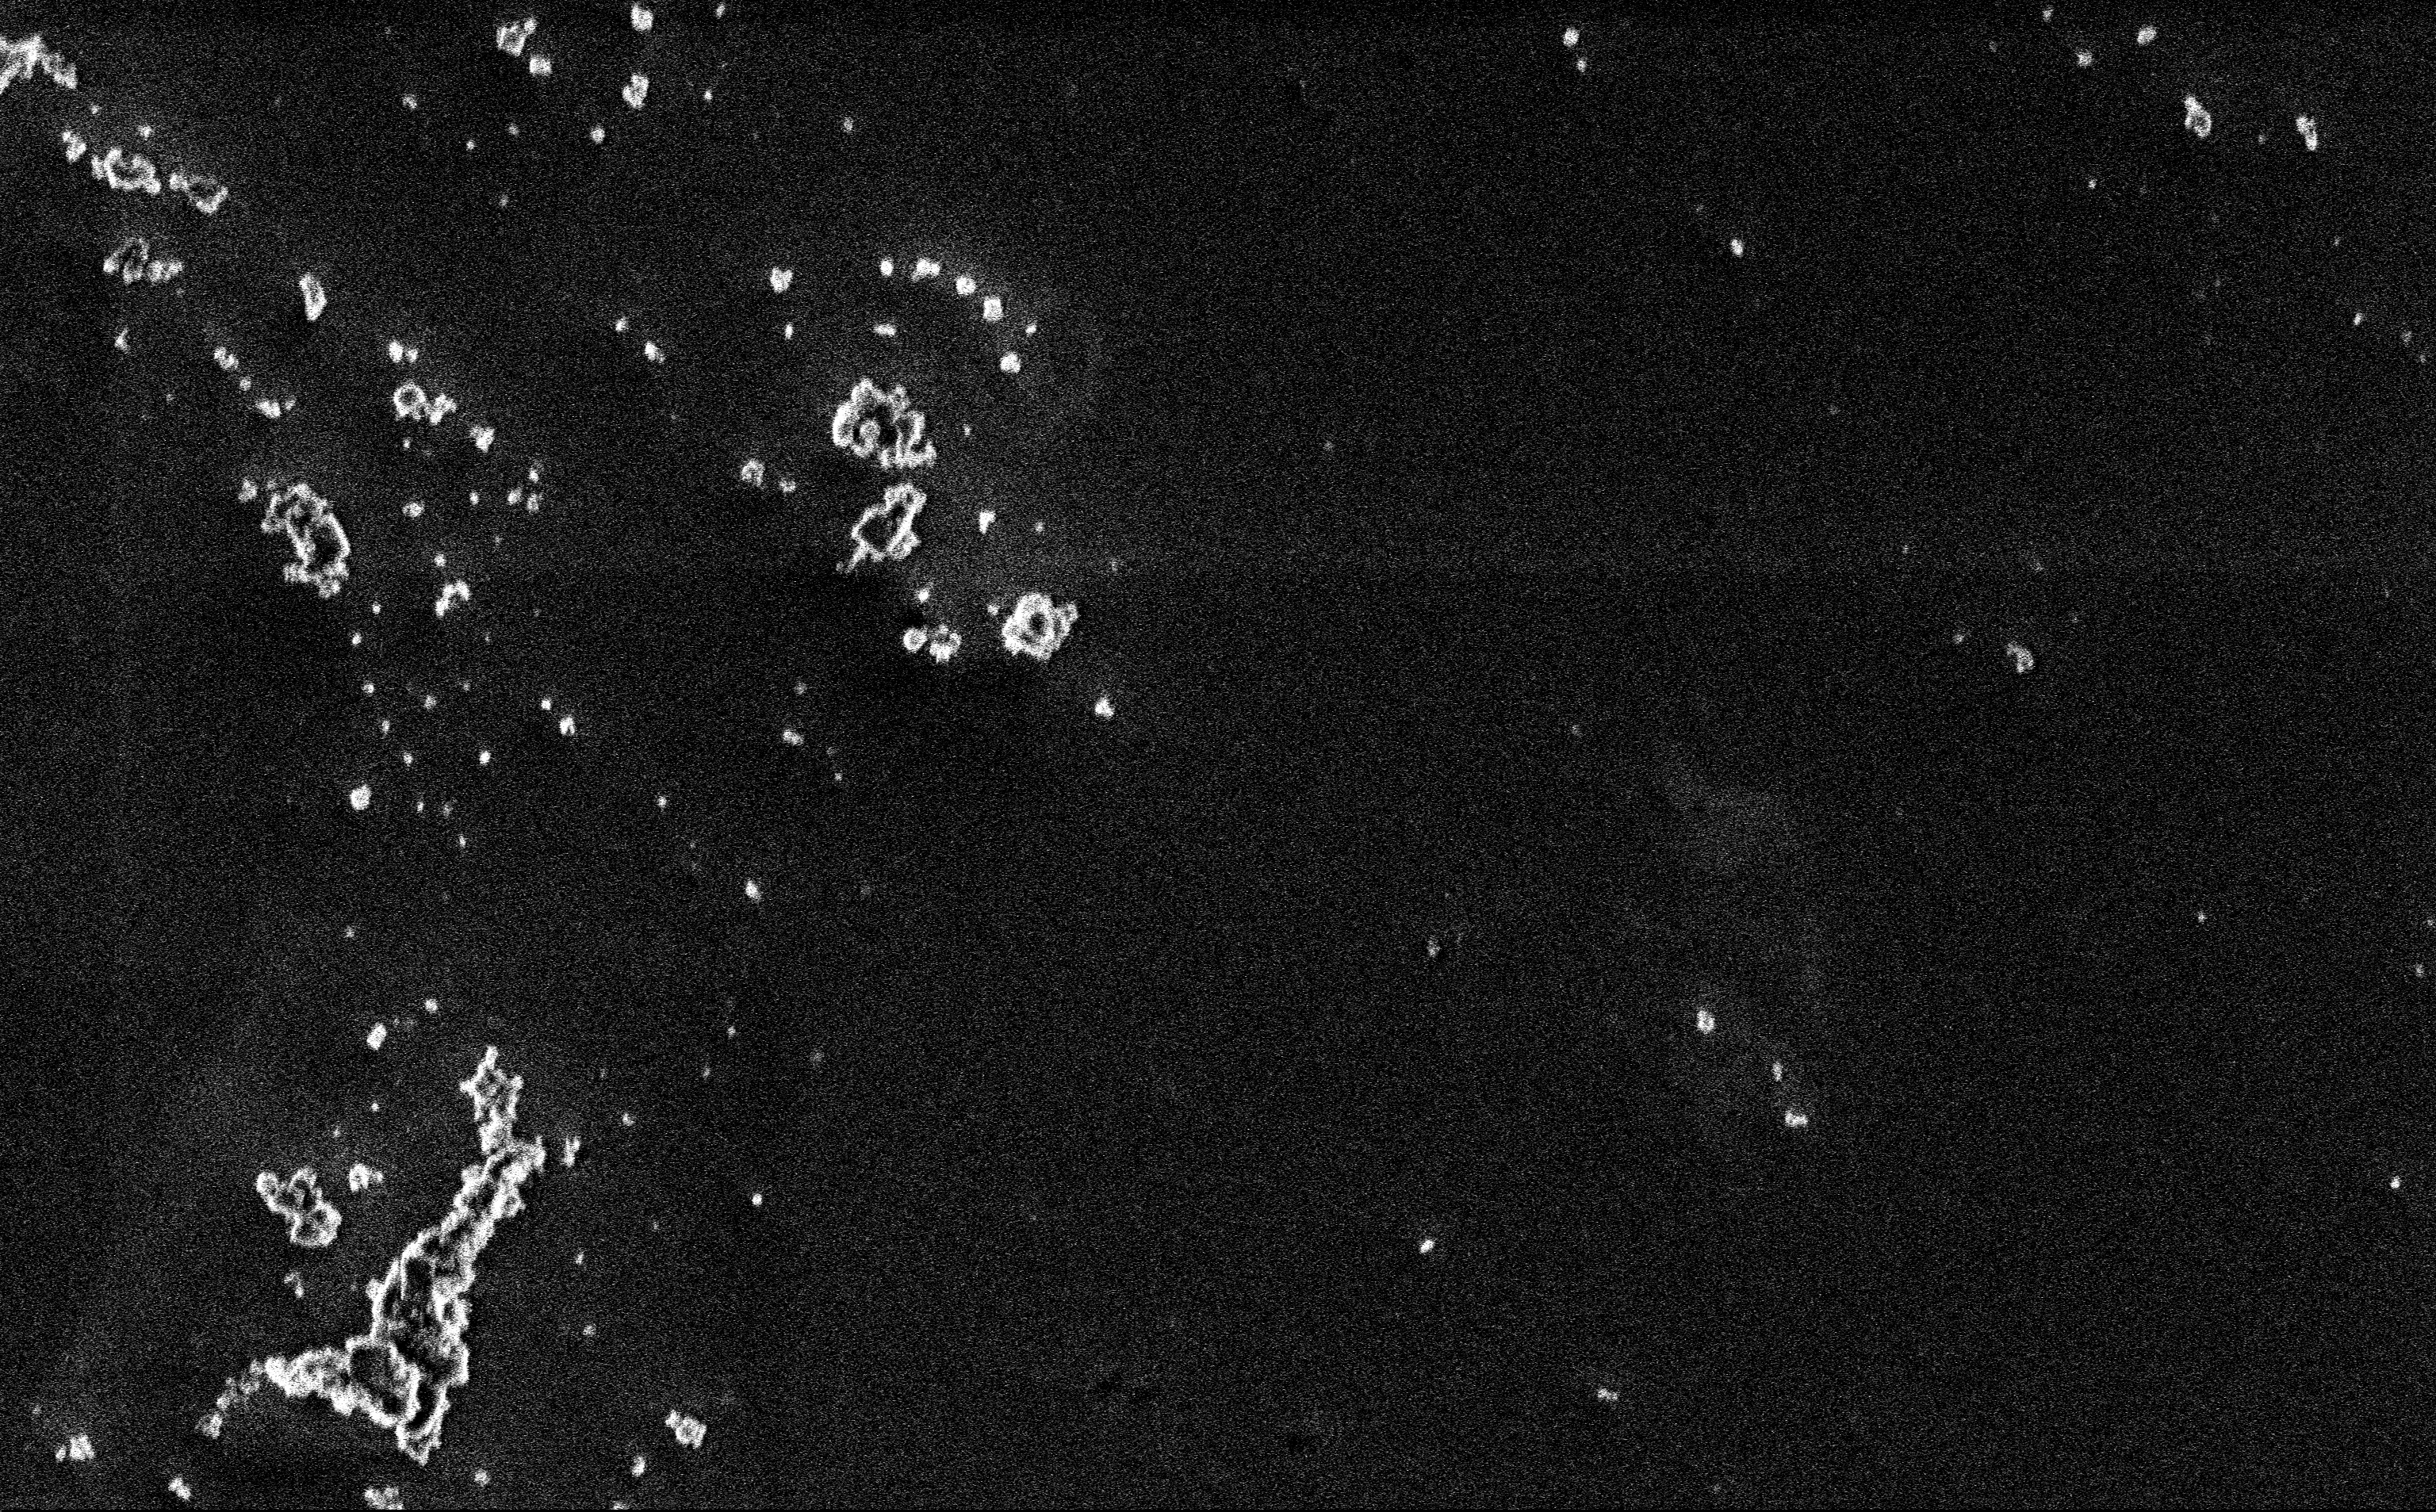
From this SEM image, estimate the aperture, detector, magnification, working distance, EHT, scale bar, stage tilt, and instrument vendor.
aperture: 30 µm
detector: InLens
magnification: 12.85 K X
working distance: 3 mm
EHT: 3 kV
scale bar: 2000 nm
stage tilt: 0°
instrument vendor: Zeiss